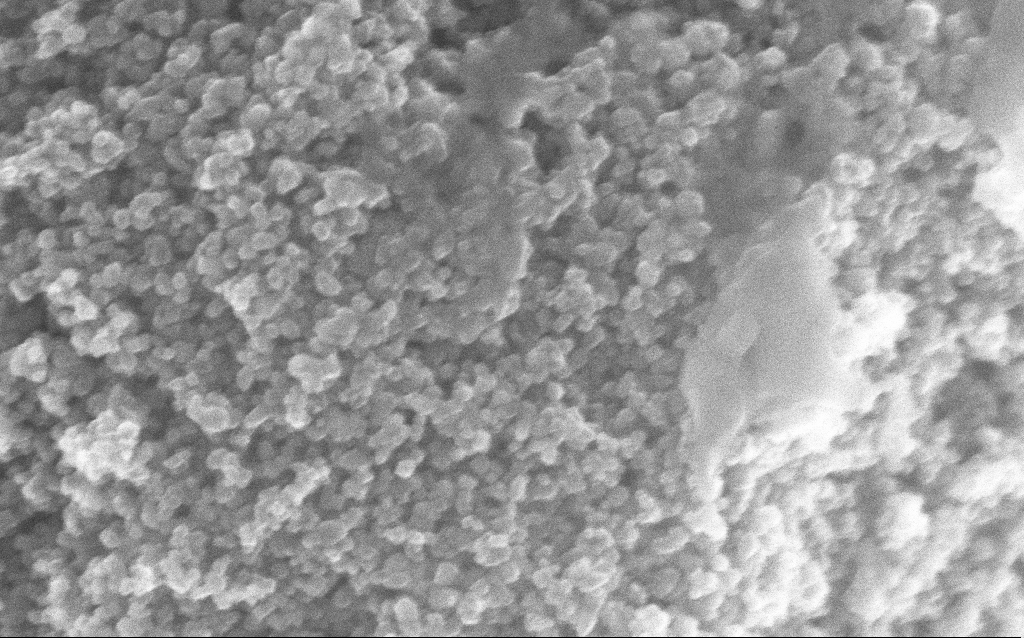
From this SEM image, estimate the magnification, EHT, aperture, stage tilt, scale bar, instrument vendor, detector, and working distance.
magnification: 239.68 K X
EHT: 10 kV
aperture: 30 µm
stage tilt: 0°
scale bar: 100 nm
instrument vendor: Zeiss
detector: InLens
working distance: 3.8 mm